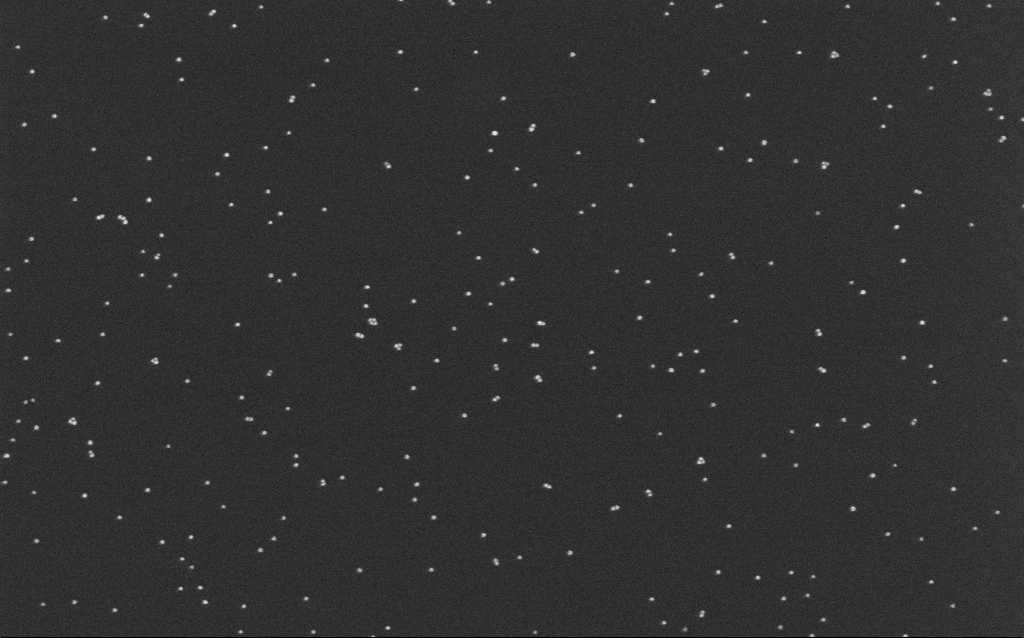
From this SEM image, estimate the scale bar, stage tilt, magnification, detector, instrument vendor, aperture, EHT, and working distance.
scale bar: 200 nm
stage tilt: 0°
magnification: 100 K X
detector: InLens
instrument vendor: Zeiss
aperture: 30 µm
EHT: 10 kV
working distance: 6.6 mm